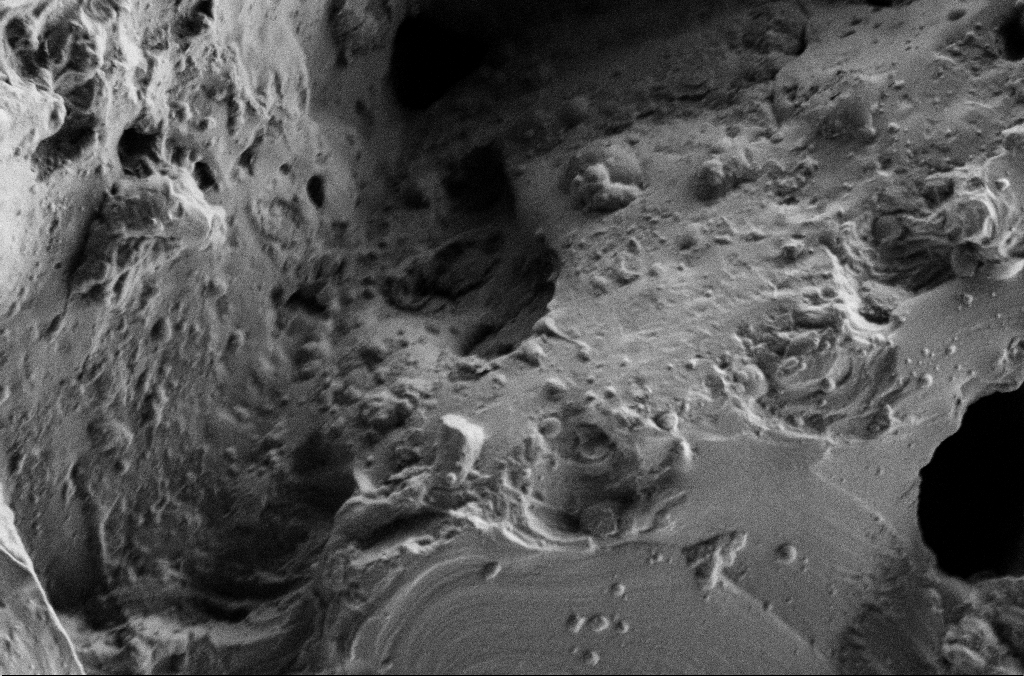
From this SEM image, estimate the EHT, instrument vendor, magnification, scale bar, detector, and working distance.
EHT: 2 kV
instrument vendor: Zeiss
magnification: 5 K X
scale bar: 10000 nm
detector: SE2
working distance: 3 mm